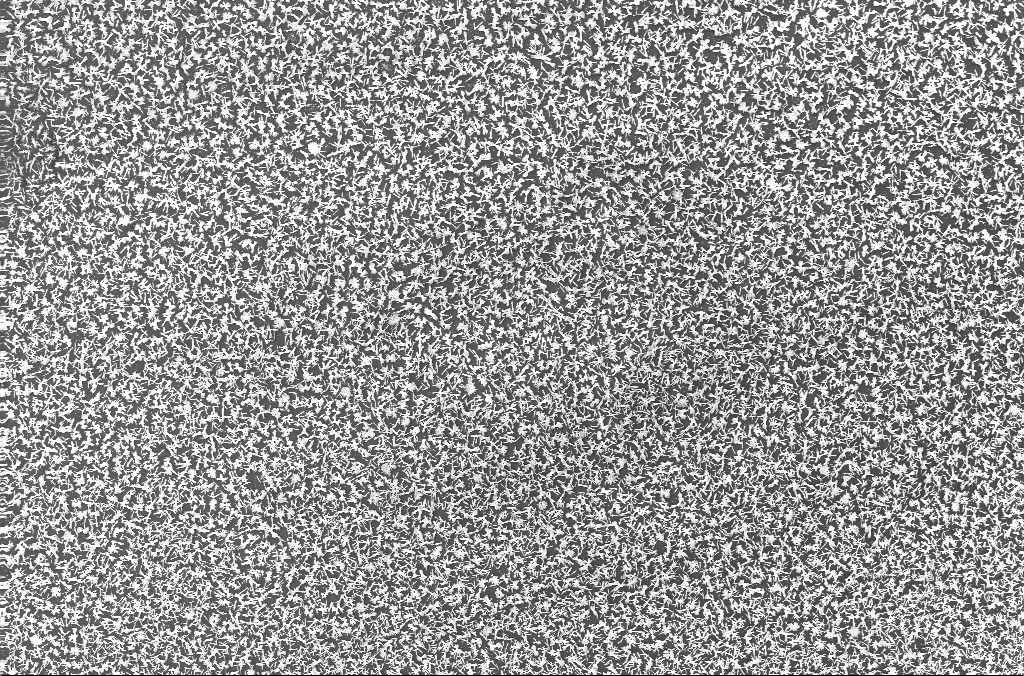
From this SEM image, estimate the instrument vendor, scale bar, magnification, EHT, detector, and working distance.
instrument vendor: Zeiss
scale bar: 100000 nm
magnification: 0.5 K X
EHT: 20 kV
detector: InLens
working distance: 2.8 mm